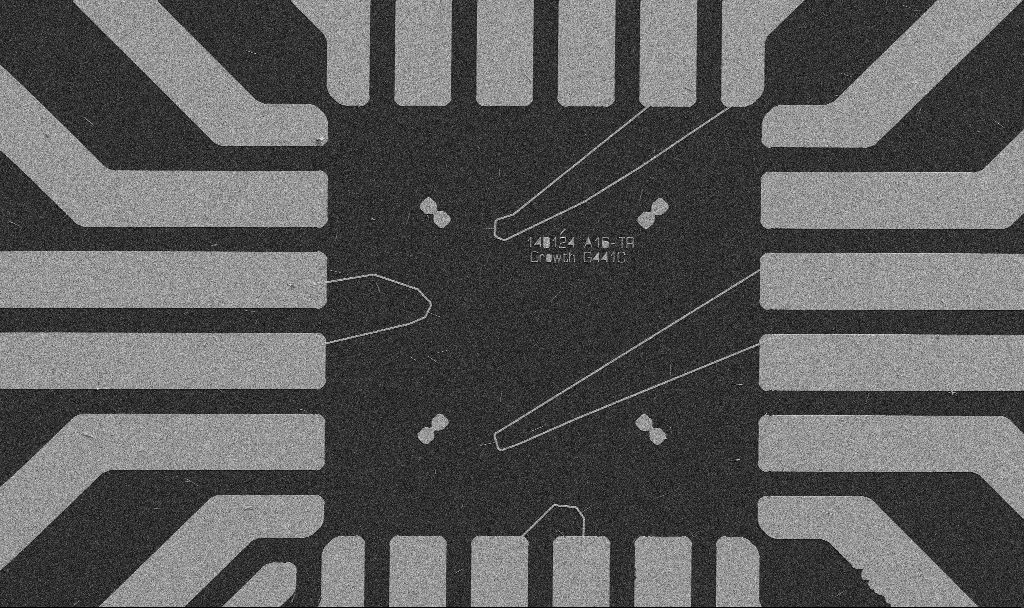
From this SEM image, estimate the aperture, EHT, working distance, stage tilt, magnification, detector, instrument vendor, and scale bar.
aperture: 30 µm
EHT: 5 kV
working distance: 10.7 mm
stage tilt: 0°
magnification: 1 K X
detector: SE2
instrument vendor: Zeiss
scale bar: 20000 nm